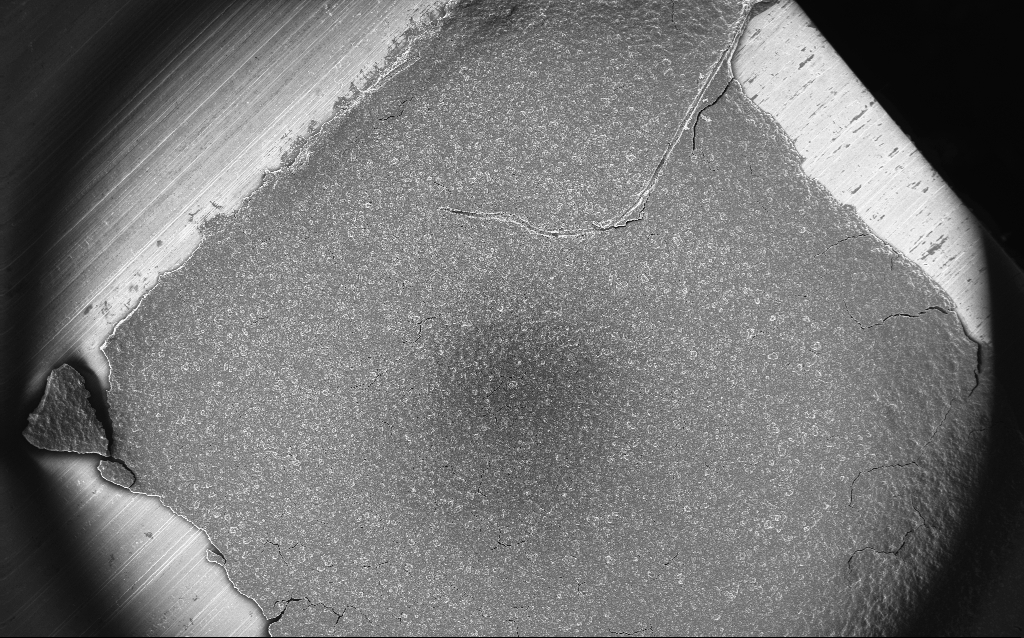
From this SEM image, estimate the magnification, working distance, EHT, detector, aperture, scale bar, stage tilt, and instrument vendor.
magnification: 0.122 K X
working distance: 2.6 mm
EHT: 10 kV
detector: InLens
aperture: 30 µm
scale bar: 100000 nm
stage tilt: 0°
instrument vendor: Zeiss